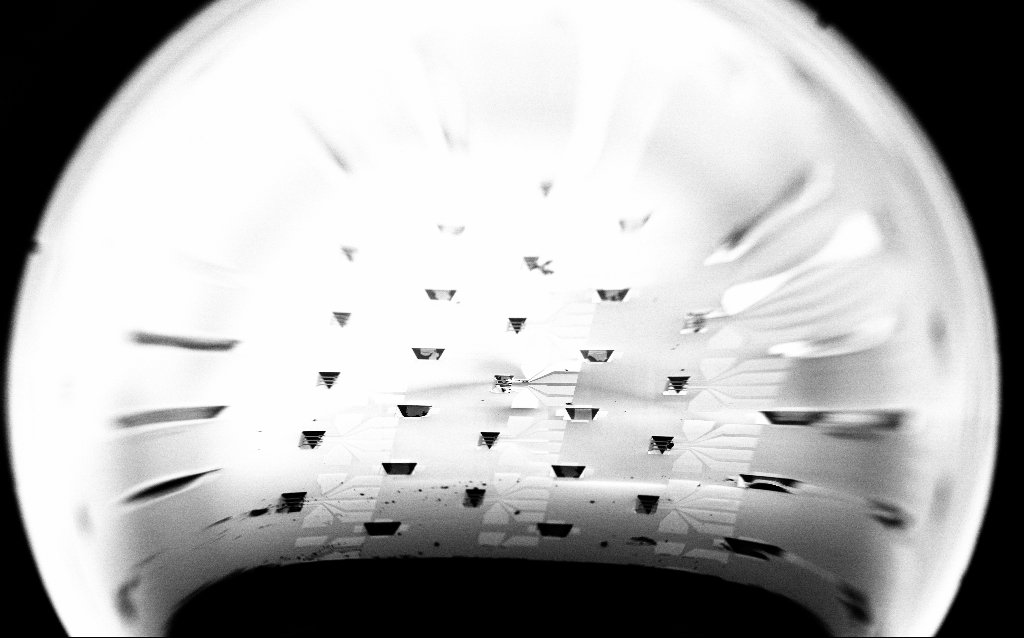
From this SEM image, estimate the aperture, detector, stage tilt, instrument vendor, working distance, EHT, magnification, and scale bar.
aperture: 30 µm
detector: SE2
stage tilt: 70°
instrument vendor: Zeiss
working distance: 8.9 mm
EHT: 2 kV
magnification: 0.061 K X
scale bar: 1e+06 nm